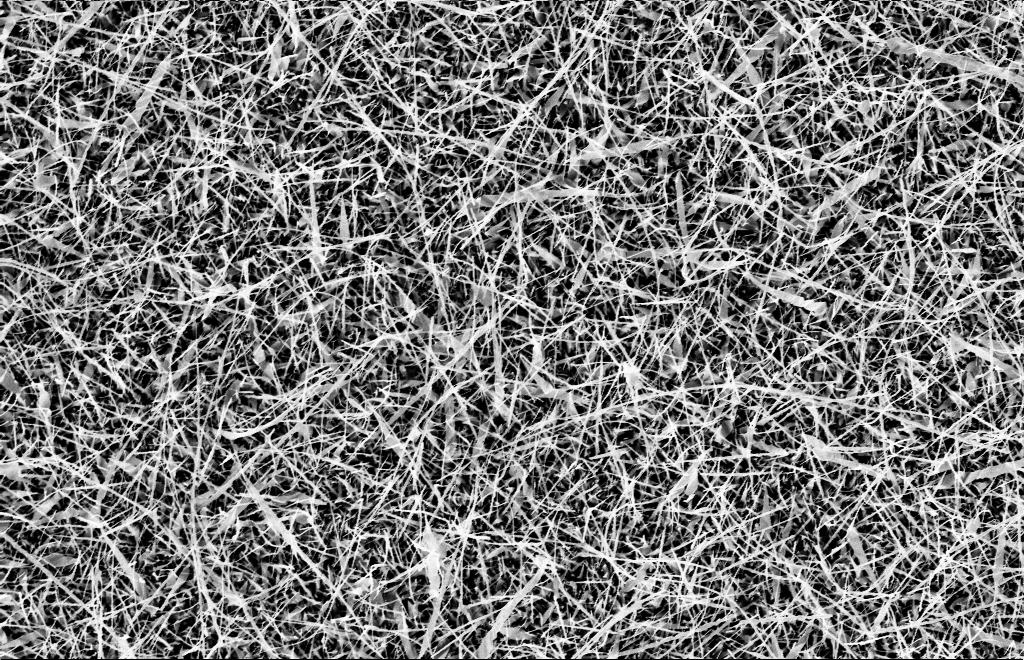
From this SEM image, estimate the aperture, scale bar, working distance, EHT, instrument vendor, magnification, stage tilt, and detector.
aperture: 20 µm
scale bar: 2000 nm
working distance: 9 mm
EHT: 10 kV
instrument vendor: Zeiss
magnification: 5 K X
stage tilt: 0°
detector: InLens